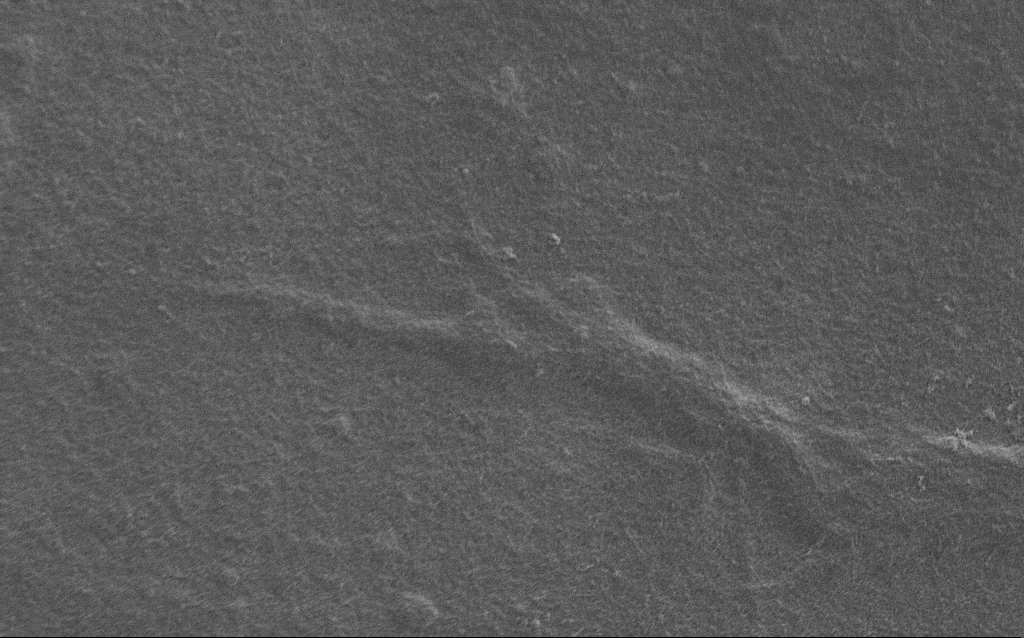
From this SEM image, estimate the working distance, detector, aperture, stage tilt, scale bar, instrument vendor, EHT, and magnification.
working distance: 6 mm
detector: SE2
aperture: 30 µm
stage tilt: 0°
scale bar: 2000 nm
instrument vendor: Zeiss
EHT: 0.9 kV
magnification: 7.5 K X